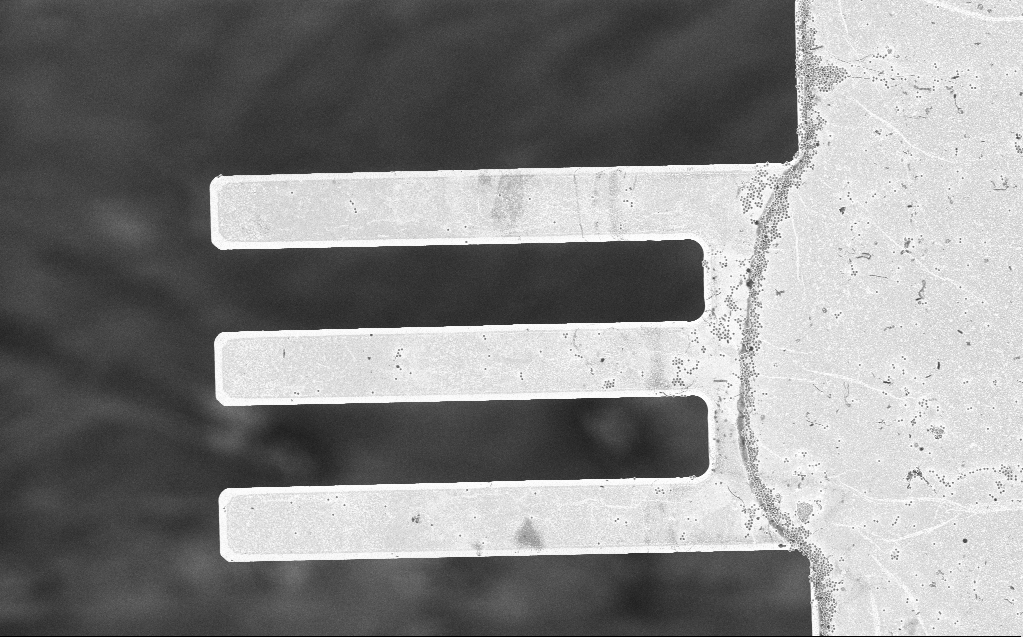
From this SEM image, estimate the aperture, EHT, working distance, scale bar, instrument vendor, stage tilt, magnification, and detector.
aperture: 30 µm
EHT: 3 kV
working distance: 7 mm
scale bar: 20000 nm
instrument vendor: Zeiss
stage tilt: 0°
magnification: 1.44 K X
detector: InLens